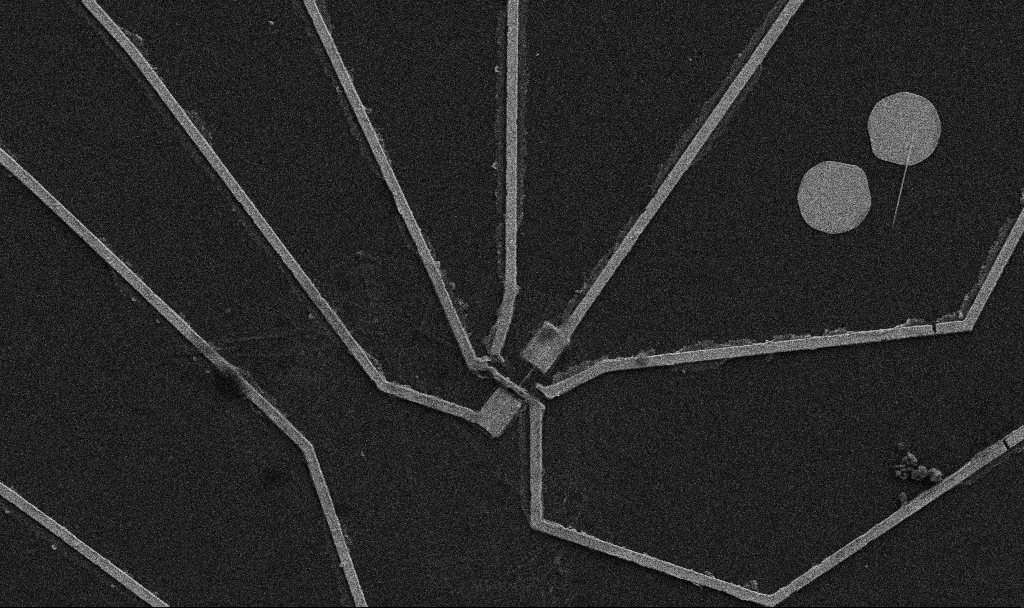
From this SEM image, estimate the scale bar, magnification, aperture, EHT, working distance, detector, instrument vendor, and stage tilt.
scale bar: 10000 nm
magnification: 5 K X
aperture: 30 µm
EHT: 5 kV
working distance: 10.7 mm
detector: SE2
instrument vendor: Zeiss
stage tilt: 0°